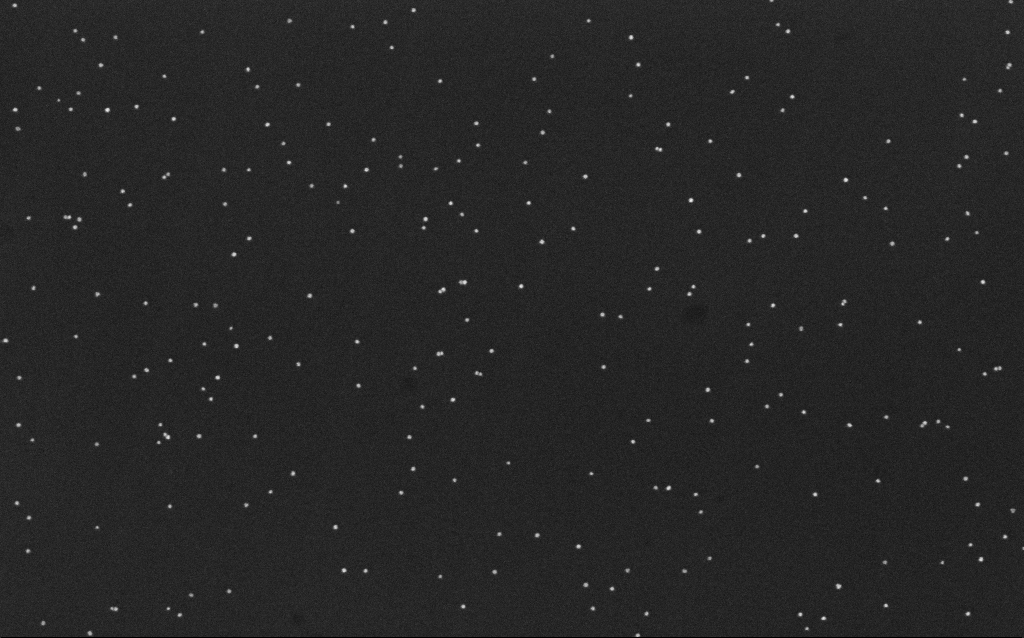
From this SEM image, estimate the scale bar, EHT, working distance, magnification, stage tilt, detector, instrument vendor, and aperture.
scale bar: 200 nm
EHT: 10 kV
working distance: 6.5 mm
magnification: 100 K X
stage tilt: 0°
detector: InLens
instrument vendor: Zeiss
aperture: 30 µm